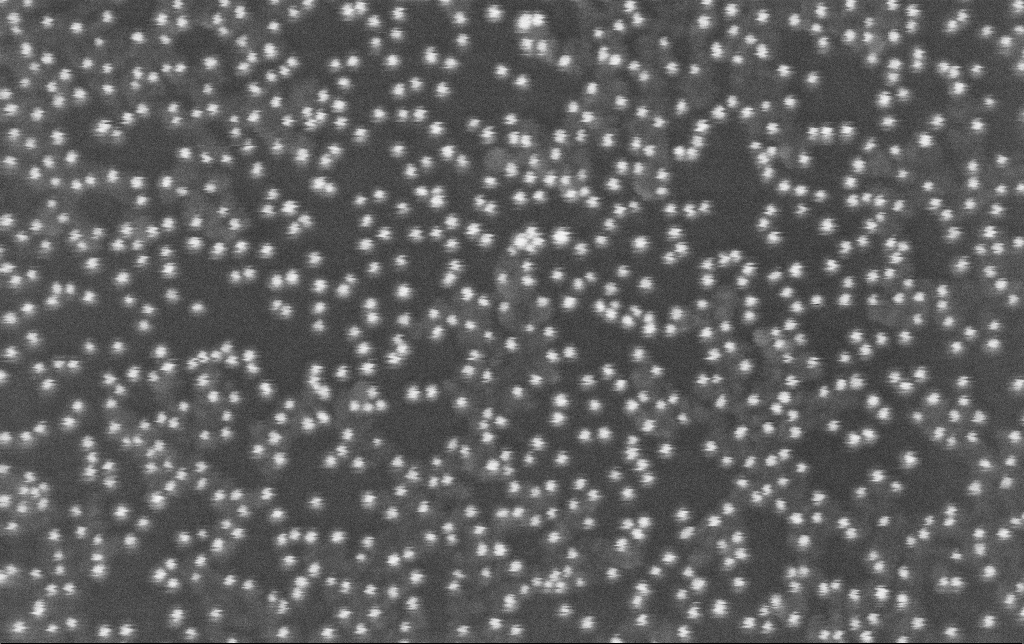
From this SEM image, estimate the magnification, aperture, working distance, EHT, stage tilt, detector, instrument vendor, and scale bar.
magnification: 282.84 K X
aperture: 30 µm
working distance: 11.3 mm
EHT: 8 kV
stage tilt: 0°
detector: InLens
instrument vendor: Zeiss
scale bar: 200 nm